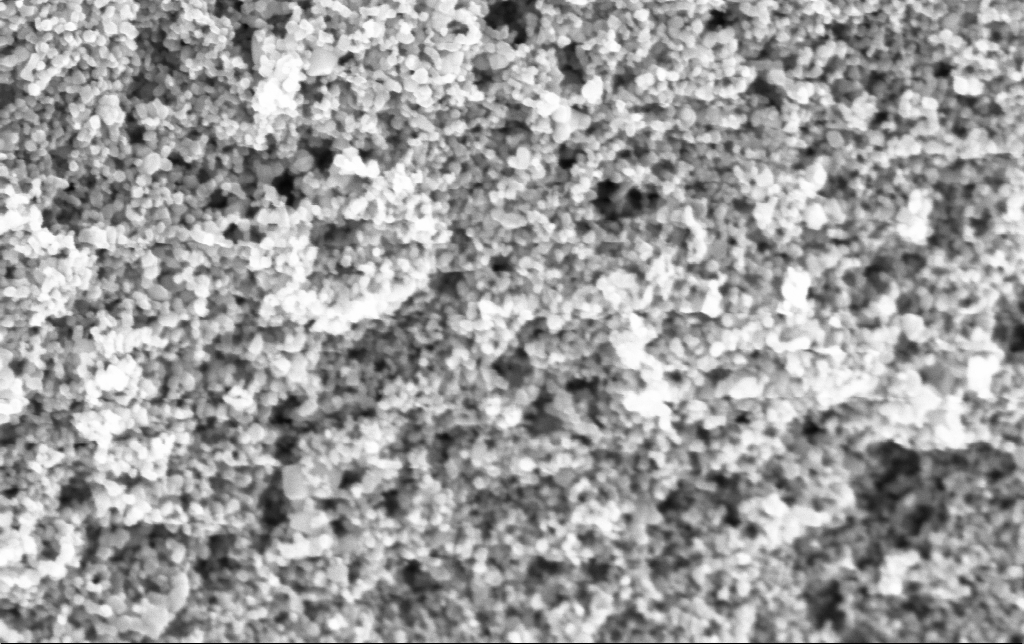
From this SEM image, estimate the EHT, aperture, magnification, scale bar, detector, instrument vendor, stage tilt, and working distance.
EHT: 5 kV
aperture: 30 µm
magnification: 135 K X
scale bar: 100 nm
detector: InLens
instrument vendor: Zeiss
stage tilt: -0°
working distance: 4.9 mm